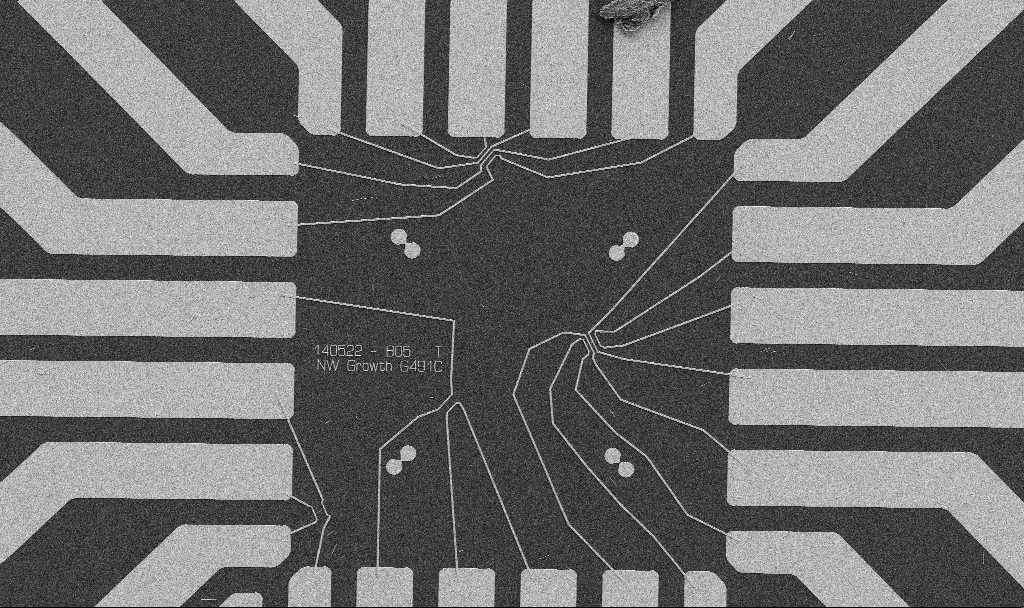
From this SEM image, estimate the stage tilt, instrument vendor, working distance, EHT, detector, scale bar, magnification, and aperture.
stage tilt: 0°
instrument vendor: Zeiss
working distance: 10.7 mm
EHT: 5 kV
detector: SE2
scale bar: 20000 nm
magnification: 1 K X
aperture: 30 µm